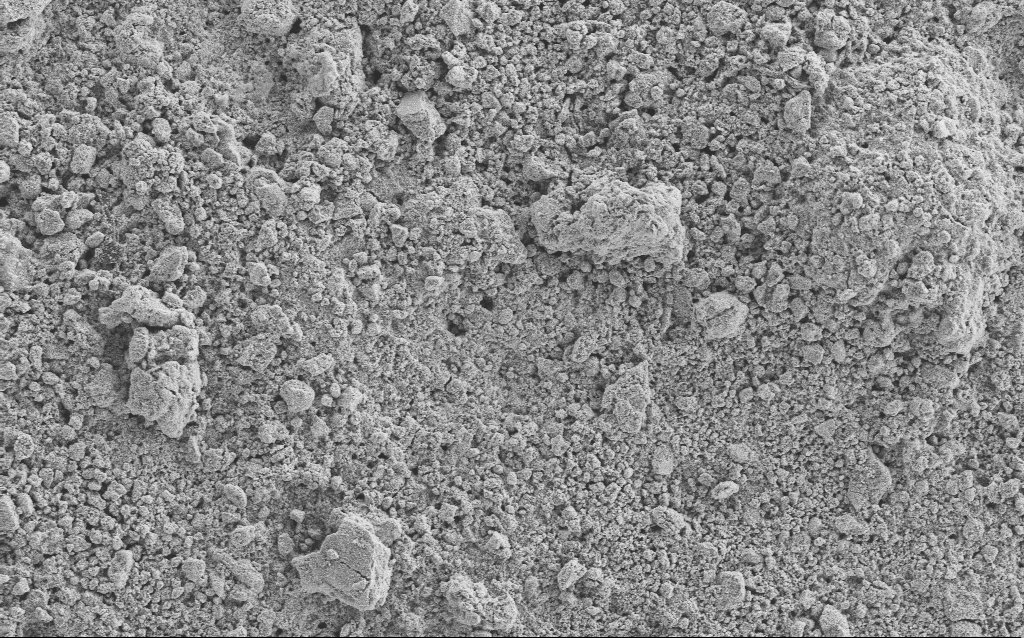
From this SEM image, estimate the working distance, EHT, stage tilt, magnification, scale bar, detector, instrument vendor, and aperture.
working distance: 4.6 mm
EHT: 5 kV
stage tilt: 0°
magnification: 1.23 K X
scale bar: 10000 nm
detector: SE2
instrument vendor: Zeiss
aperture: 30 µm